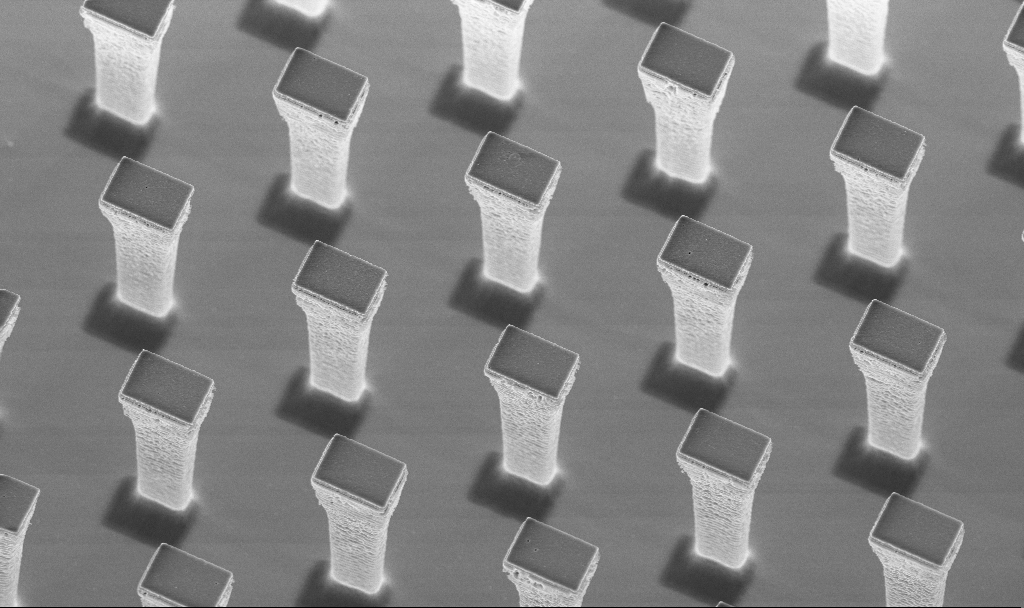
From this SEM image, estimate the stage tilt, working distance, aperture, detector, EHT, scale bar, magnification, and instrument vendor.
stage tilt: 20°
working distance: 4.2 mm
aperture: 30 µm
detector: InLens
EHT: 5 kV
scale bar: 10000 nm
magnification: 6.51 K X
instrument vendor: Zeiss